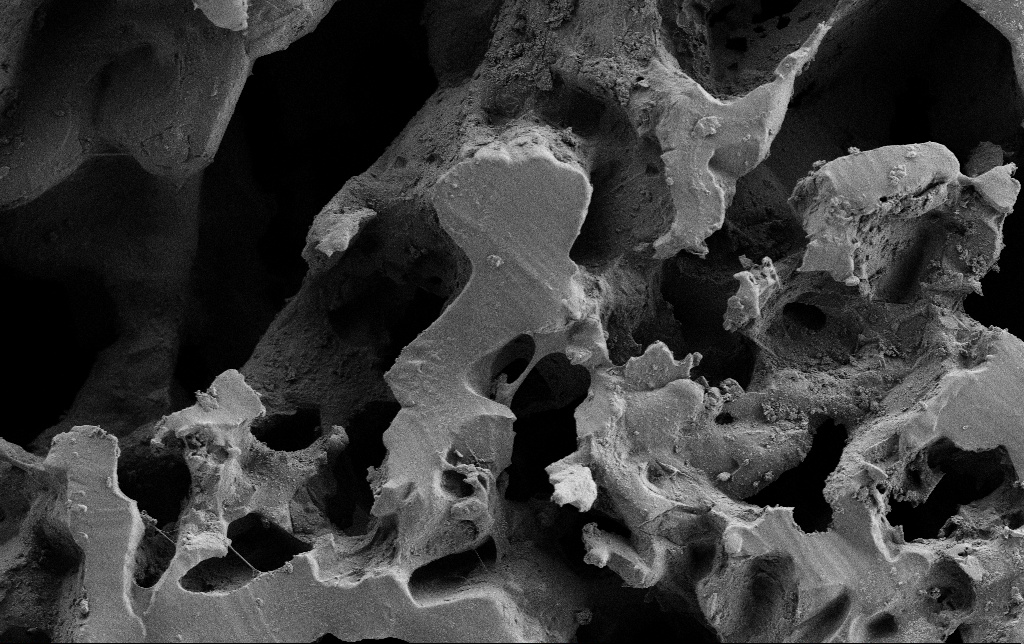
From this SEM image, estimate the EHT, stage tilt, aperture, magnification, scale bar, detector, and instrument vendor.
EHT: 3 kV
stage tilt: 0°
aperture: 30 µm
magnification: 1 K X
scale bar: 20000 nm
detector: SE2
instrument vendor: Zeiss